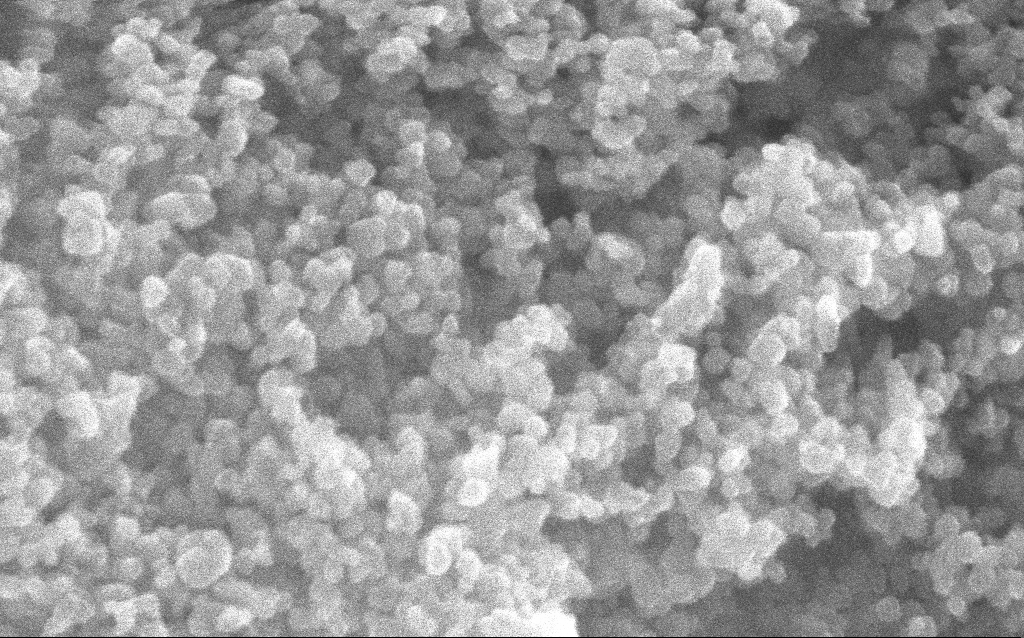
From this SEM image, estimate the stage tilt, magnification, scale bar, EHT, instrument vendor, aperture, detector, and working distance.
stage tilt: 0°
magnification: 348.1 K X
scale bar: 100 nm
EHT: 10 kV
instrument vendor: Zeiss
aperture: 30 µm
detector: InLens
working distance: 2.4 mm